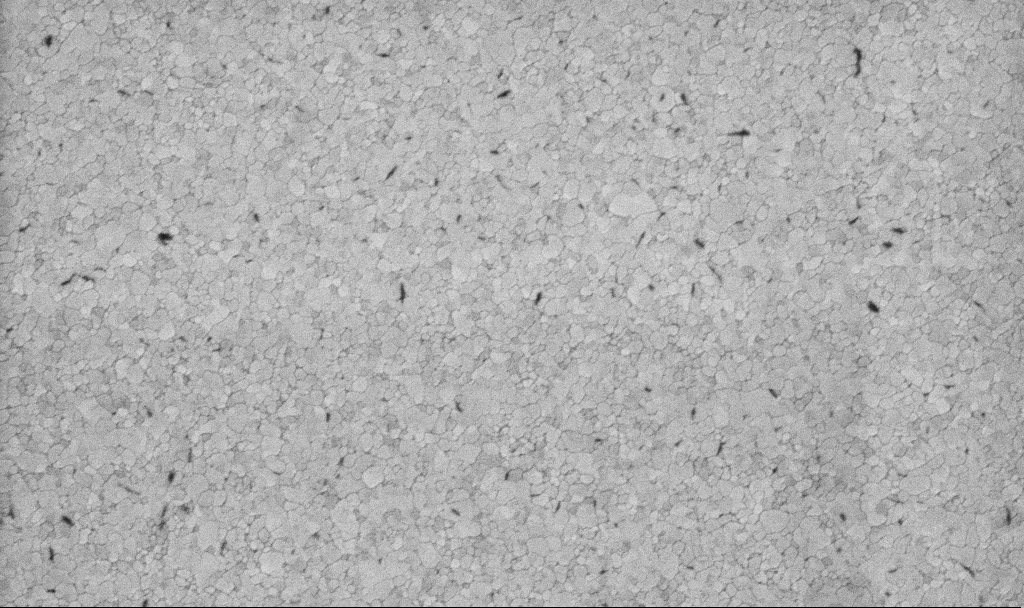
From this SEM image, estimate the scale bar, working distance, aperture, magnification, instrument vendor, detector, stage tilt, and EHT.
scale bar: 1000 nm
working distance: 3.1 mm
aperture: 30 µm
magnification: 50.15 K X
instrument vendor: Zeiss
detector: InLens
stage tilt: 0°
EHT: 5 kV